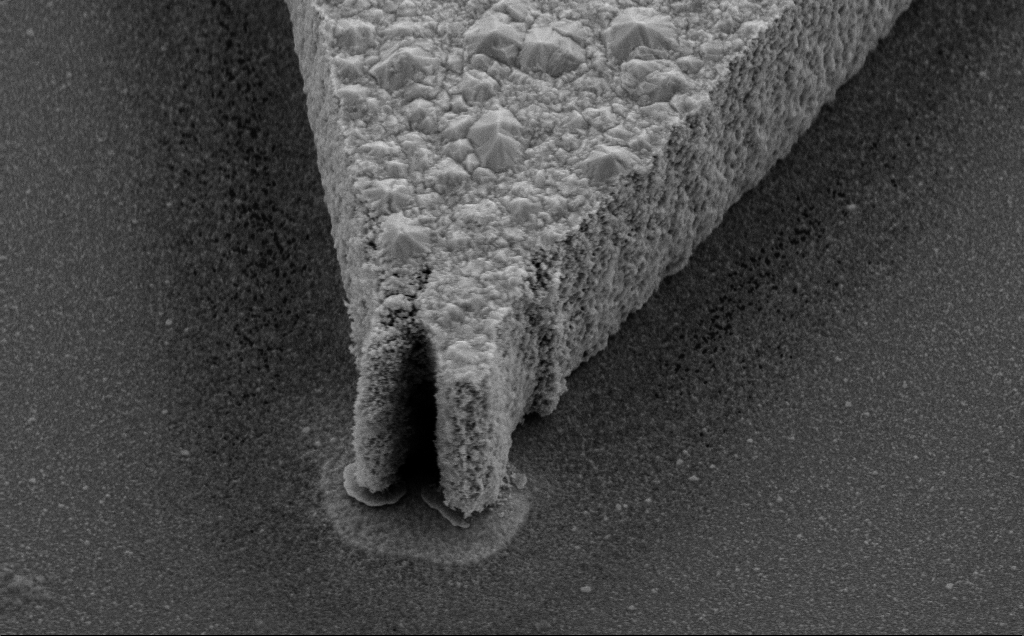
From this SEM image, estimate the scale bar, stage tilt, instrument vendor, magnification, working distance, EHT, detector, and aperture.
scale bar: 2000 nm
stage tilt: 35°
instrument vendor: Zeiss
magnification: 19.35 K X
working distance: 8 mm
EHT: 10 kV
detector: SE2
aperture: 30 µm